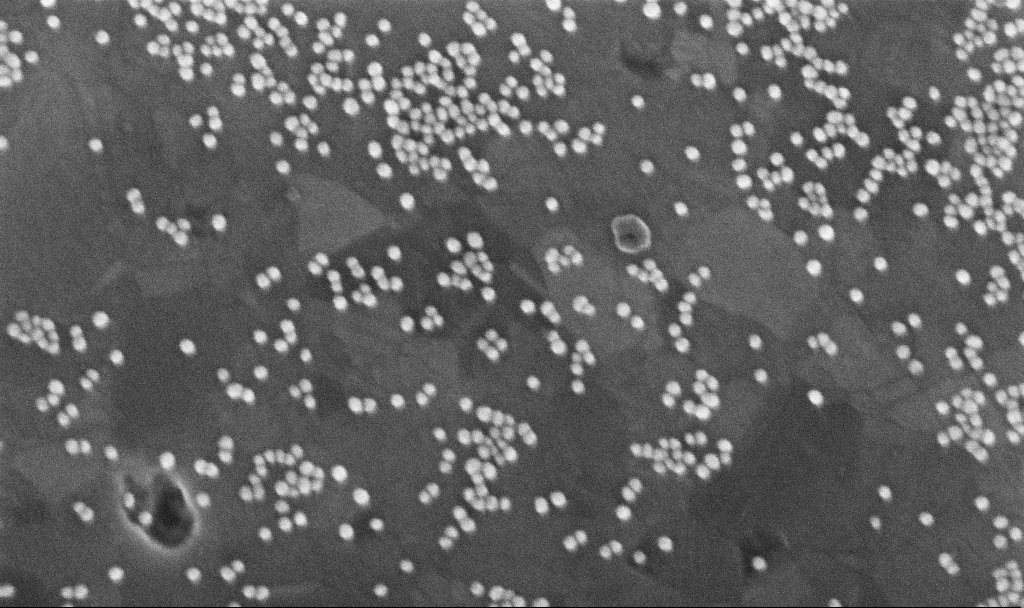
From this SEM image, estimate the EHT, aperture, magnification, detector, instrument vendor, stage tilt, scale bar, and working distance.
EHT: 10 kV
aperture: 30 µm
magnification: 254.91 K X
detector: InLens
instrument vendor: Zeiss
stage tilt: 0°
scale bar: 200 nm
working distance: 3.7 mm